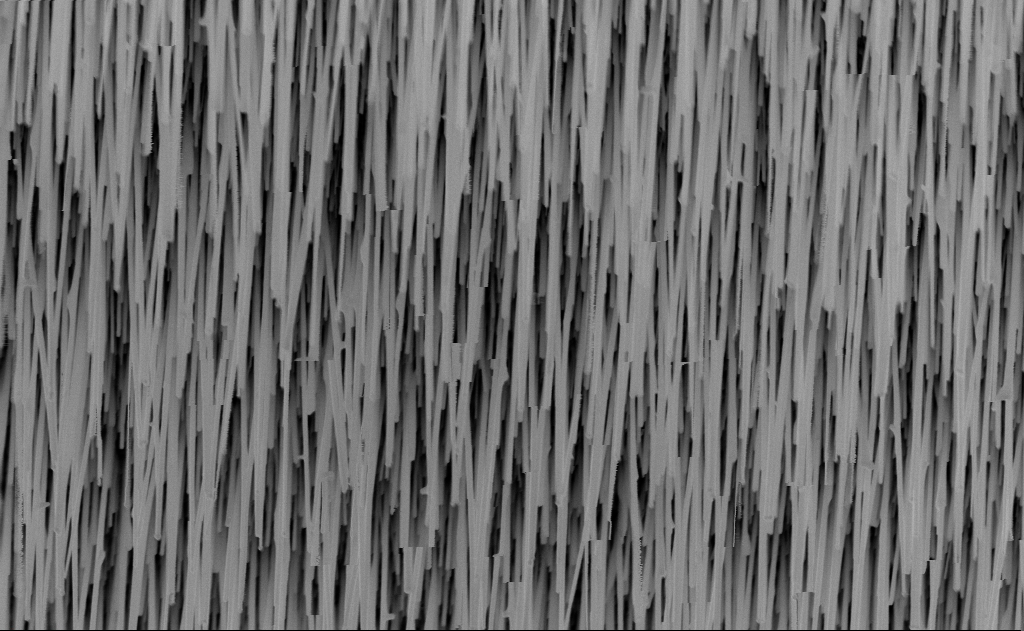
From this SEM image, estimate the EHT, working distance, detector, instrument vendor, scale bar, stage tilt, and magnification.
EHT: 10 kV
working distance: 9 mm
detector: InLens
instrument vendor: Zeiss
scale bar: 2000 nm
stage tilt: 0°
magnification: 20 K X